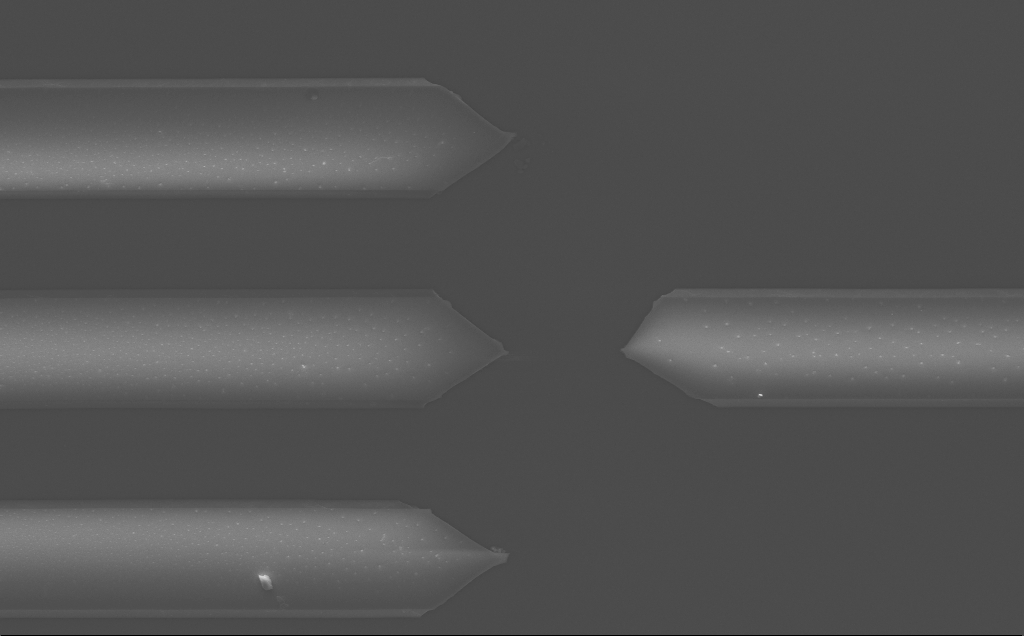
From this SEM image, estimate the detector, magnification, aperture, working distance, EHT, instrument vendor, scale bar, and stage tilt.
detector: InLens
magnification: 1.96 K X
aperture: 30 µm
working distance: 10 mm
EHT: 10 kV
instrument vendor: Zeiss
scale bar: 20000 nm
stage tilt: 0°